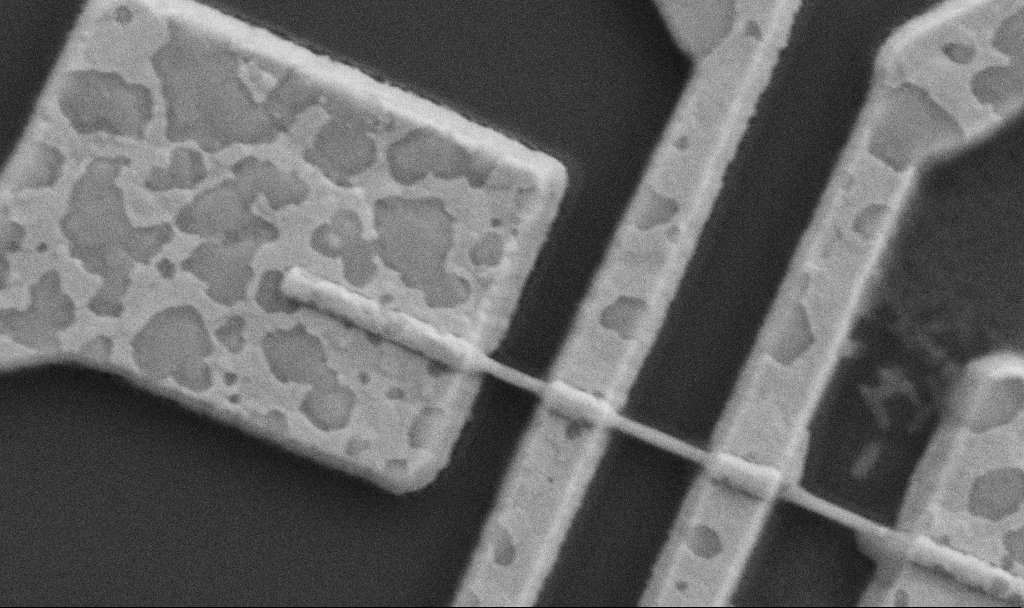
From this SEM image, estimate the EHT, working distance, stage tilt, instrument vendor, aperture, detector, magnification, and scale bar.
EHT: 5 kV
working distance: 8.7 mm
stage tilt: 0°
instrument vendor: Zeiss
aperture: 30 µm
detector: SE2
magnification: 60 K X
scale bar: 1000 nm